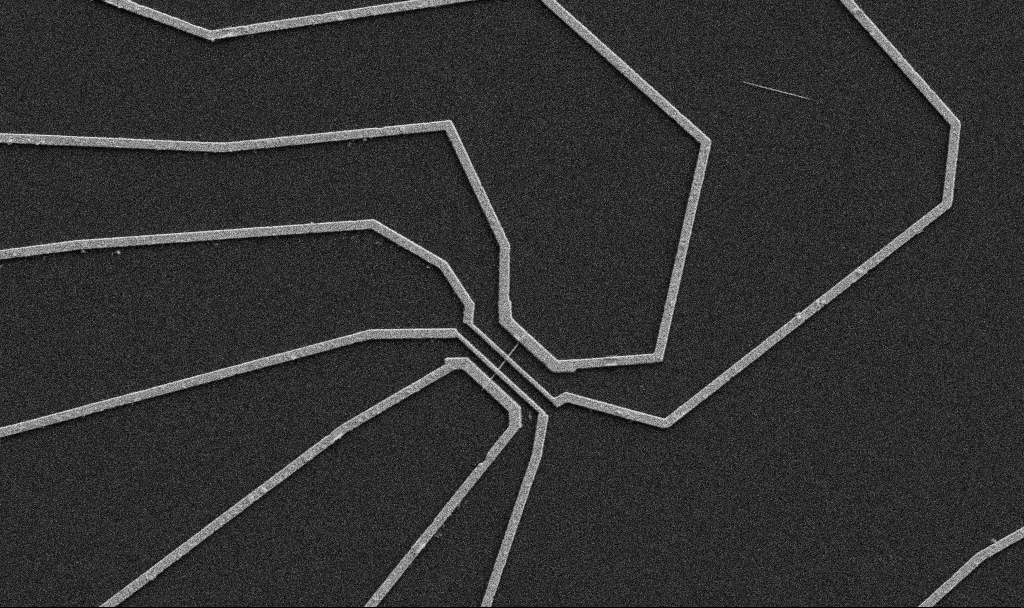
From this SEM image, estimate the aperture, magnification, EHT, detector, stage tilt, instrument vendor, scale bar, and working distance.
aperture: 30 µm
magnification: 5 K X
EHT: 5 kV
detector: SE2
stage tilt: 0°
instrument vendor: Zeiss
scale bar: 10000 nm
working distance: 10.7 mm